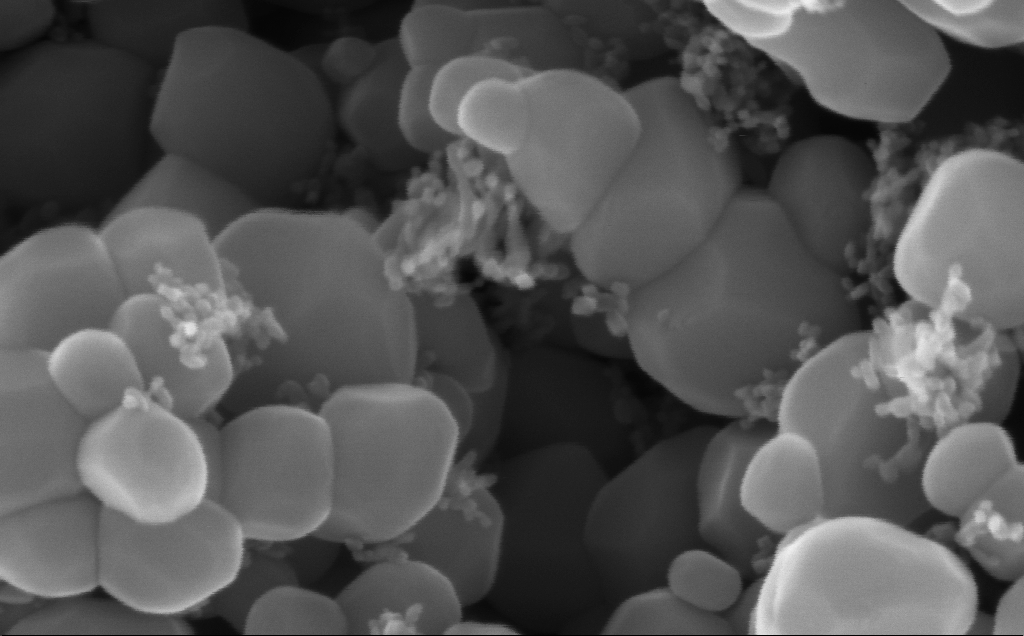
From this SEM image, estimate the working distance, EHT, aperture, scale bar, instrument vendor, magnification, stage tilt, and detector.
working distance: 2.3 mm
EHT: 5 kV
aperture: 30 µm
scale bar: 100 nm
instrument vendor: Zeiss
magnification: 416 K X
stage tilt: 0°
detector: InLens